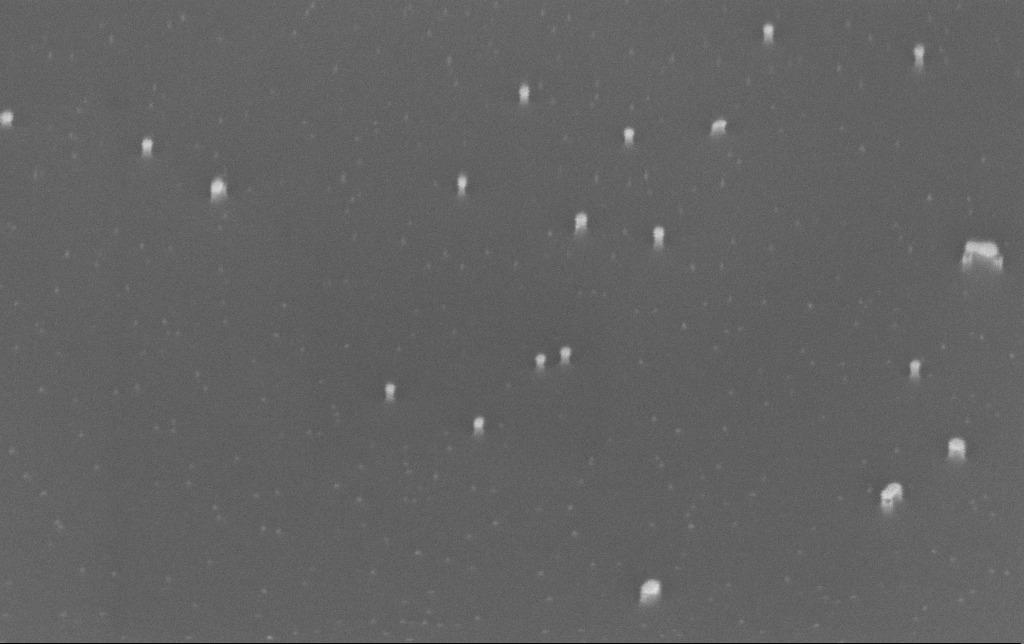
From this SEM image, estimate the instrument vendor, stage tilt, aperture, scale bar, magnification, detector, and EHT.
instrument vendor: Zeiss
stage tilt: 45°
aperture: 30 µm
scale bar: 100 nm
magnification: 200 K X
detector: InLens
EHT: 10 kV